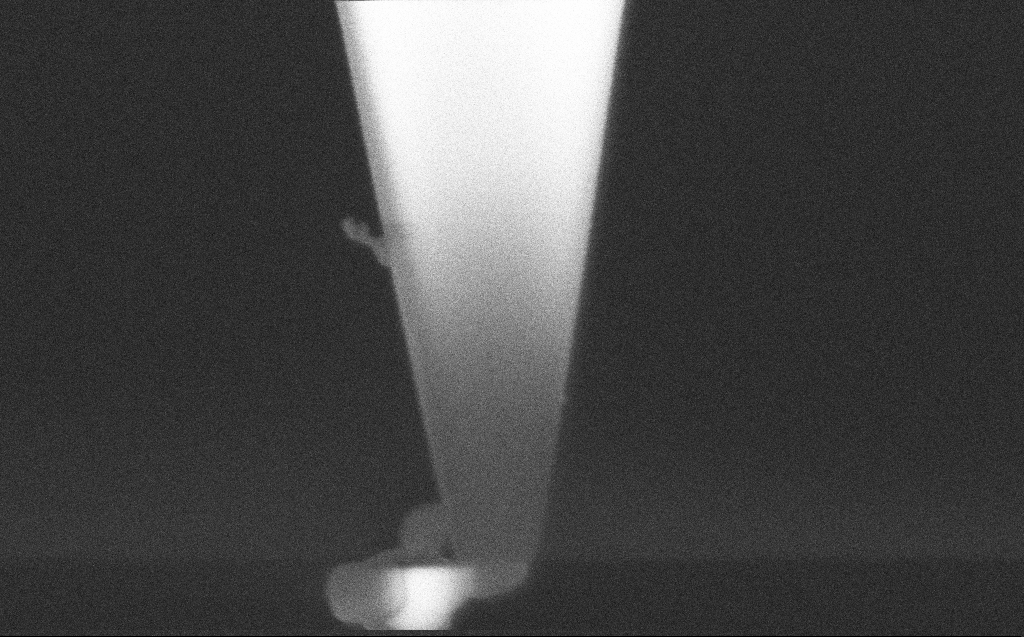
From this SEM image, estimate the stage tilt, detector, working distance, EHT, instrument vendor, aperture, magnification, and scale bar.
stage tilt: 37.3°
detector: InLens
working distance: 5 mm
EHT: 3 kV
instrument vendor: Zeiss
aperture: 30 µm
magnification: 195.01 K X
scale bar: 200 nm